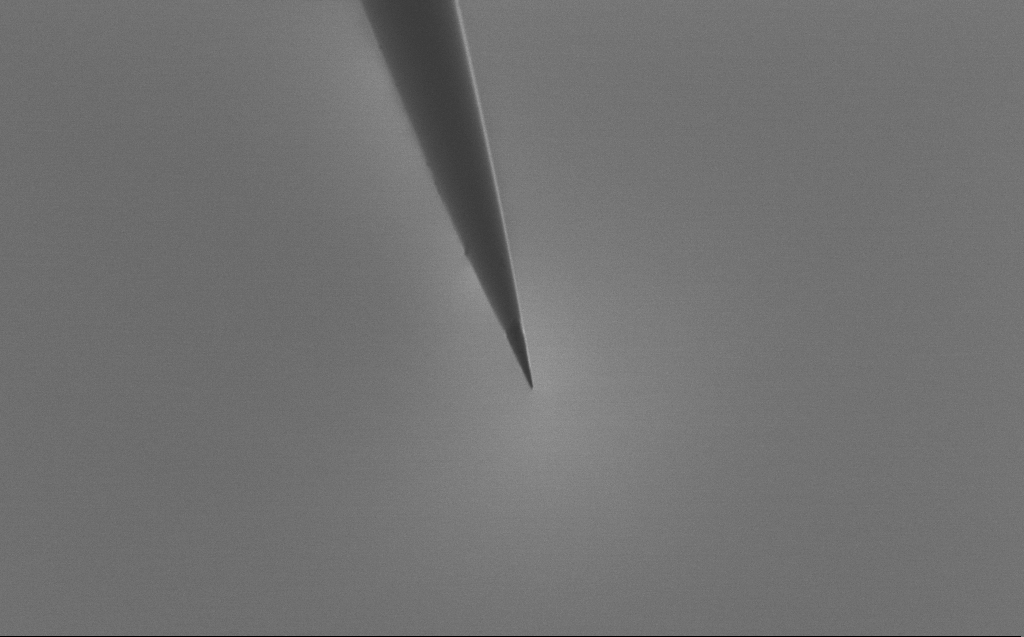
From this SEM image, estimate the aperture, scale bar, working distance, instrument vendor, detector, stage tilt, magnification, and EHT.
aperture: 30 µm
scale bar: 2000 nm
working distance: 5 mm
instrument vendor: Zeiss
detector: SE2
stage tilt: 39.3°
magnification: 10 K X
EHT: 0.8 kV